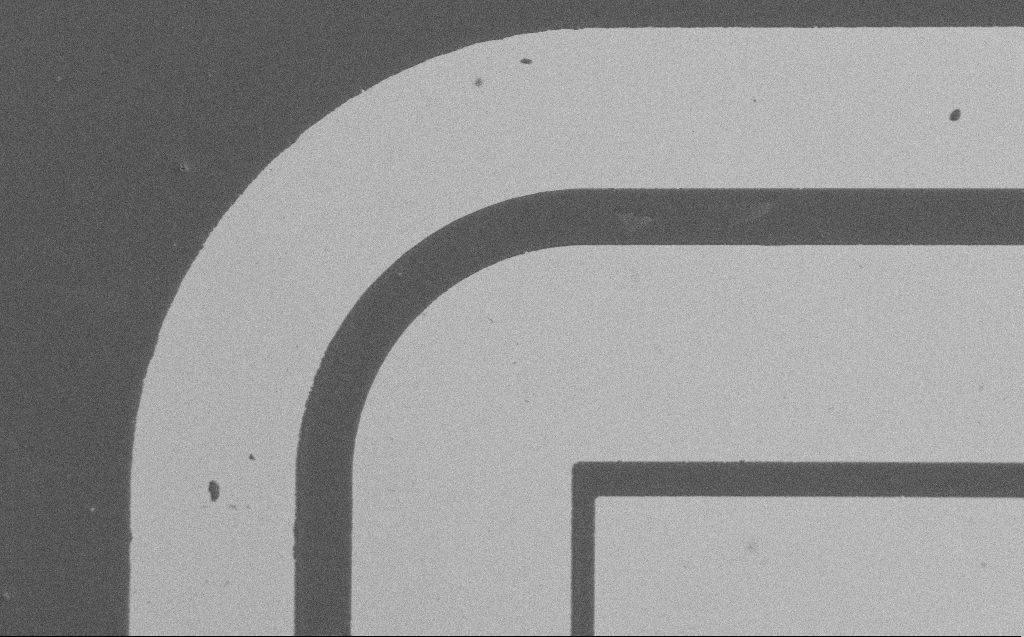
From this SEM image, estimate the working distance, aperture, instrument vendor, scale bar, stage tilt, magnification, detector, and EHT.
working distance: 6 mm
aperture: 30 µm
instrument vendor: Zeiss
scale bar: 20000 nm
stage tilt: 0°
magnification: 0.803 K X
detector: SE2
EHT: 1.2 kV